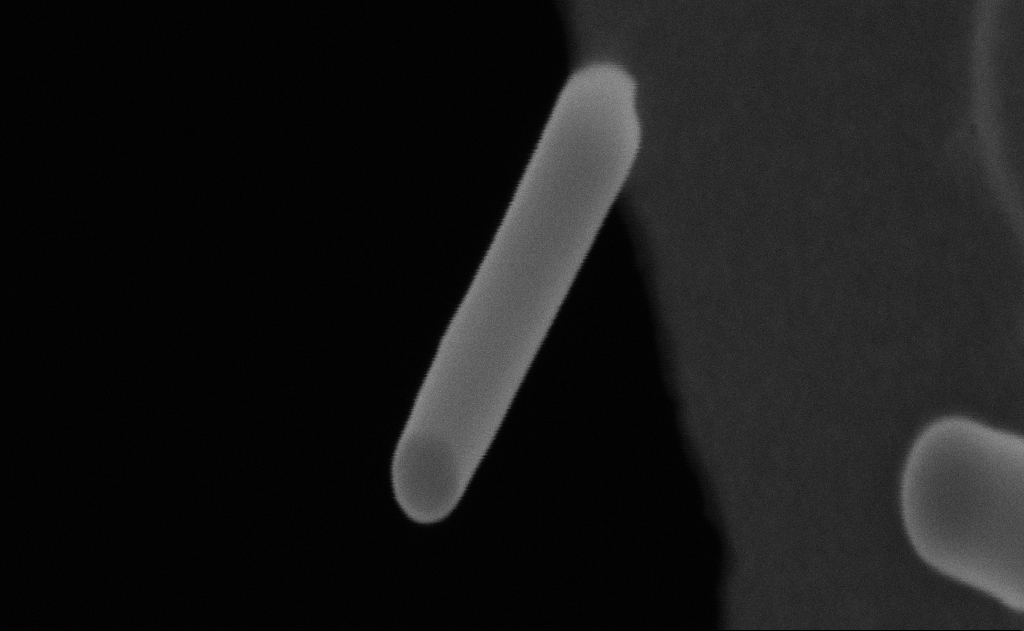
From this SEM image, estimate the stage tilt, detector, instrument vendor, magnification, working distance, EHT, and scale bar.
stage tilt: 0°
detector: SE2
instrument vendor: Zeiss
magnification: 471.42 K X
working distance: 9 mm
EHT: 20 kV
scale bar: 100 nm